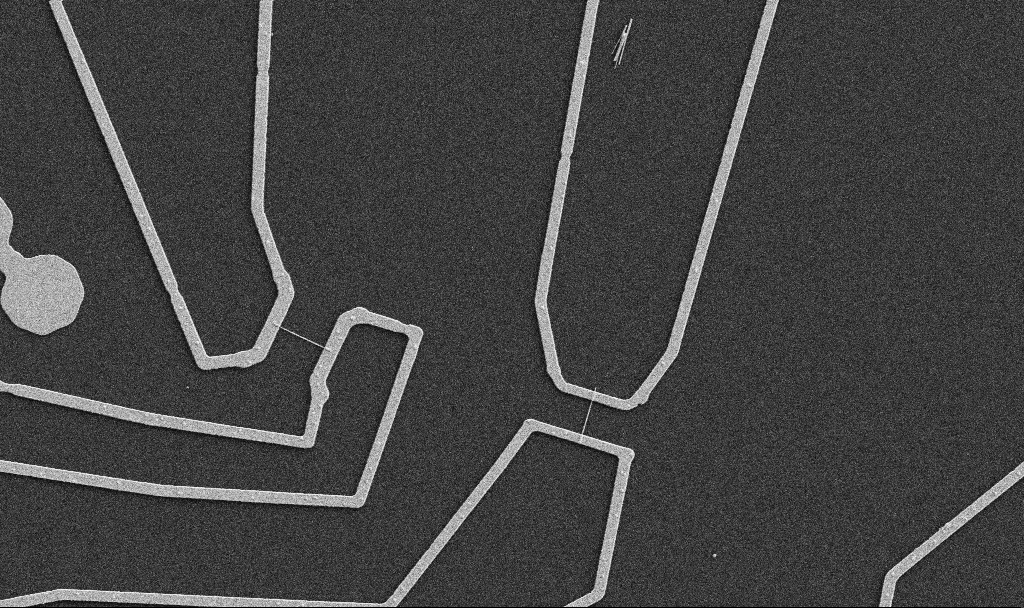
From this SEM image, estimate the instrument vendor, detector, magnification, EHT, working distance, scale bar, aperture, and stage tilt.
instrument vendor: Zeiss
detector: SE2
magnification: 5 K X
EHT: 5 kV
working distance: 10.7 mm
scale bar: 10000 nm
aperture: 30 µm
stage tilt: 0°